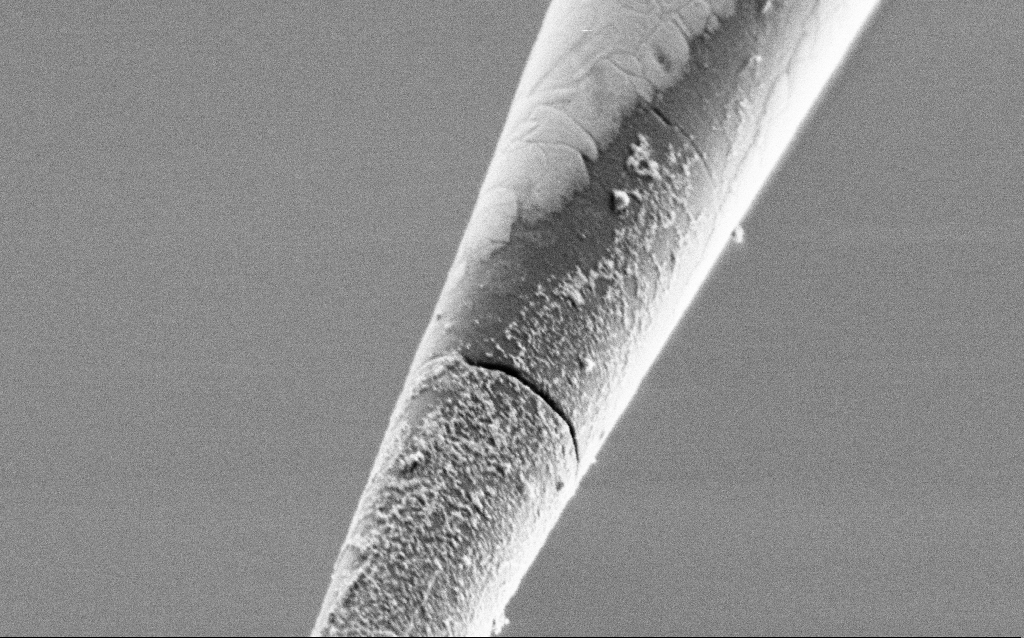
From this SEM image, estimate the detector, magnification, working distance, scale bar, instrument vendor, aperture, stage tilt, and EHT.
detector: SE2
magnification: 50 K X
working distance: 6.7 mm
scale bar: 1000 nm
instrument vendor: Zeiss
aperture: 30 µm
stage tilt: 45°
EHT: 1 kV